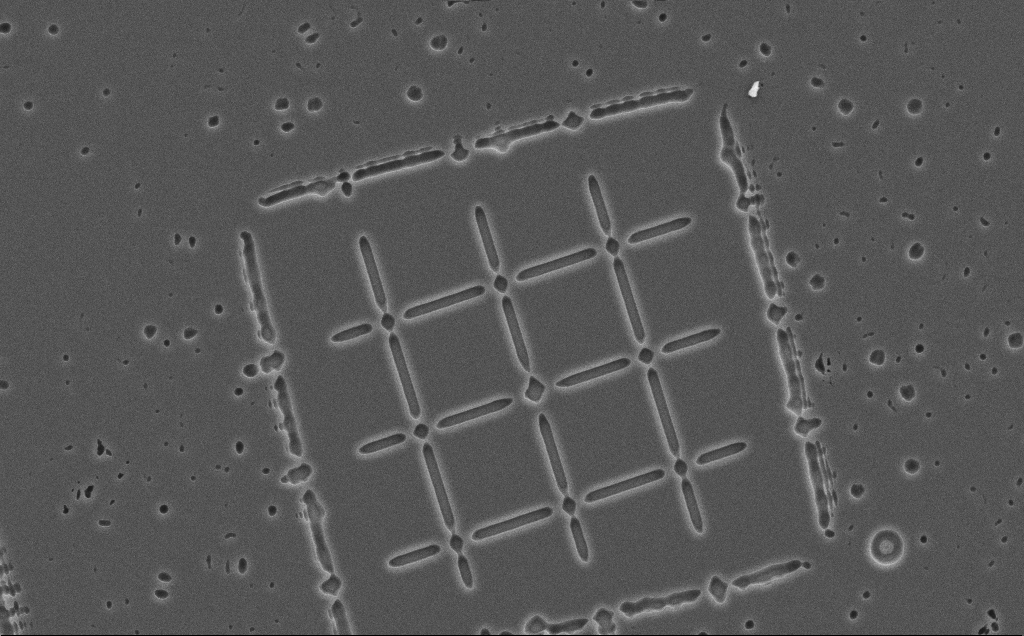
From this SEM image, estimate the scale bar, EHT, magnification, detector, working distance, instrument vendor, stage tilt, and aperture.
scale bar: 10000 nm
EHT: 10 kV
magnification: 2.13 K X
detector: SE2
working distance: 12 mm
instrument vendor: Zeiss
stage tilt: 0°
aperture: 30 µm